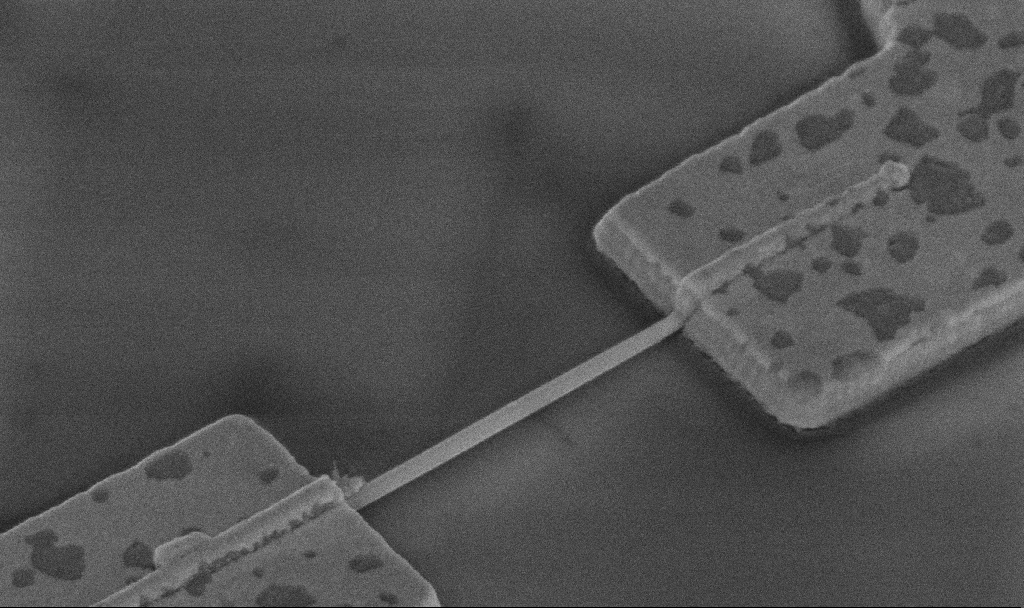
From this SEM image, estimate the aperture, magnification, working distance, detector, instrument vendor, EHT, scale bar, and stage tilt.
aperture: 30 µm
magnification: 60 K X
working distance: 13.6 mm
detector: InLens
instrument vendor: Zeiss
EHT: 5 kV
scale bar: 1000 nm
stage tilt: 45°